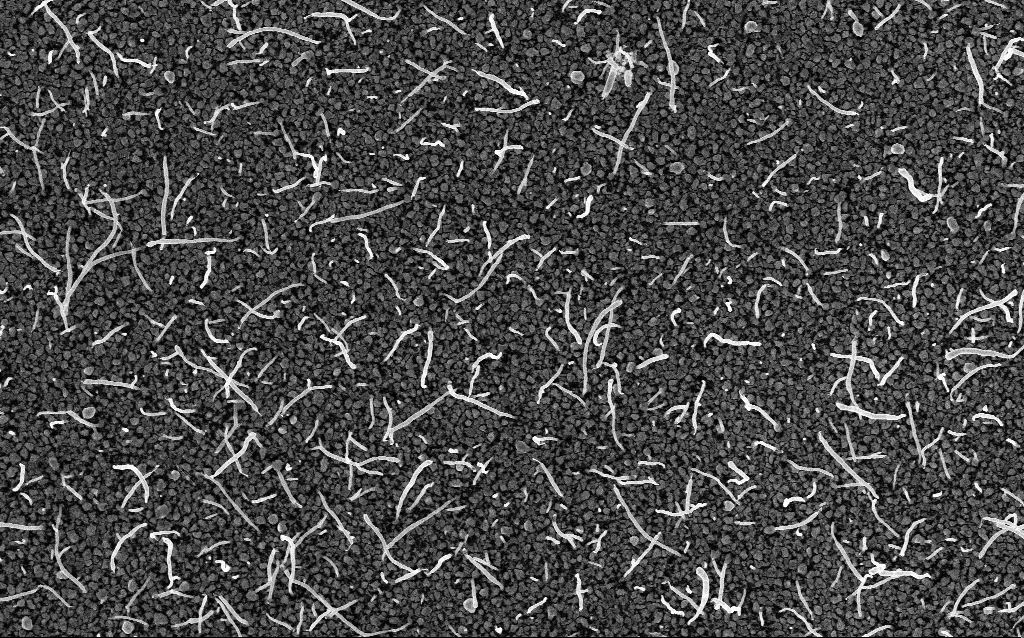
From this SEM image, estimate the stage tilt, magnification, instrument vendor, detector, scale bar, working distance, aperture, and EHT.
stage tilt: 0°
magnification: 20 K X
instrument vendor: Zeiss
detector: InLens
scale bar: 1000 nm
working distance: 2.2 mm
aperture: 30 µm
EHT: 5 kV